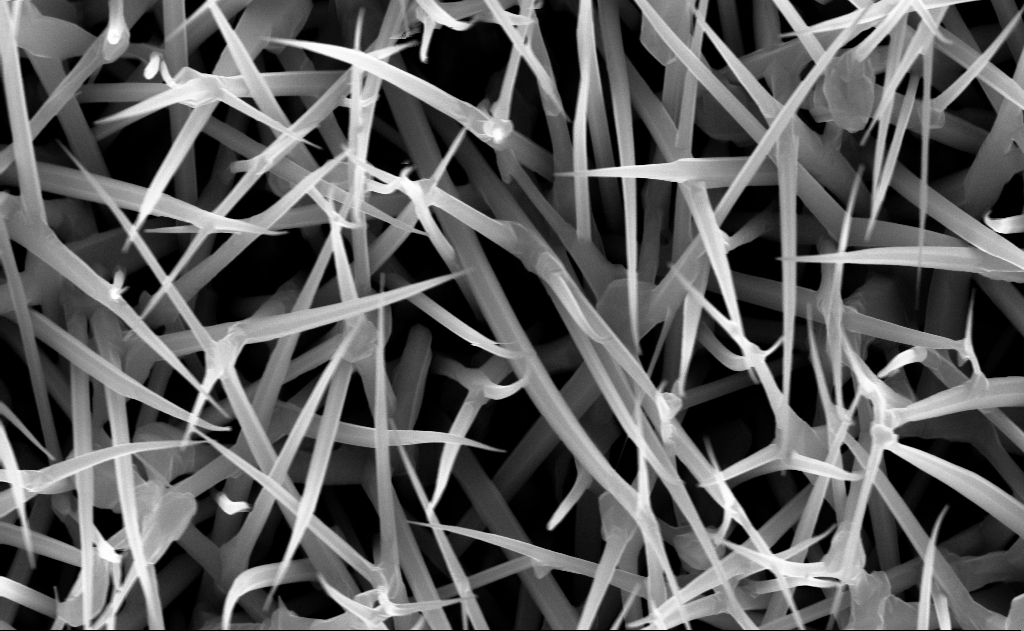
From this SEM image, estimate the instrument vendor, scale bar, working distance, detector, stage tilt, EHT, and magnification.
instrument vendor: Zeiss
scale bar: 1000 nm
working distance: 7 mm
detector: InLens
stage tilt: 0°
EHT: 10 kV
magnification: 40 K X